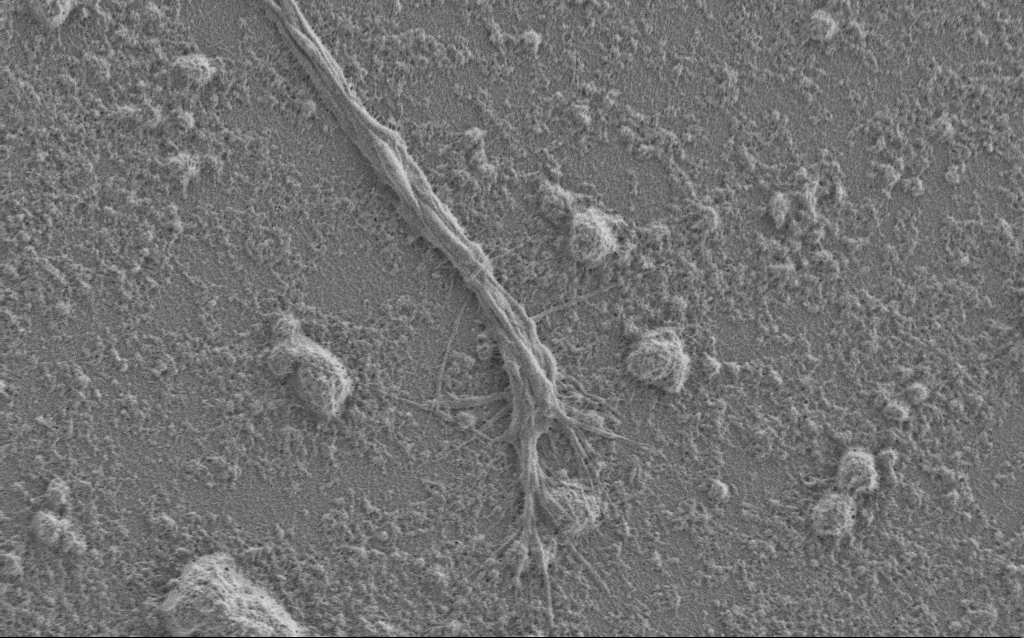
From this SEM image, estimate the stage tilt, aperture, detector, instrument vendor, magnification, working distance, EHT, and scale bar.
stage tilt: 0°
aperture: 30 µm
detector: SE2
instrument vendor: Zeiss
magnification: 7.5 K X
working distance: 6 mm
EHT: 1 kV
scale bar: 2000 nm